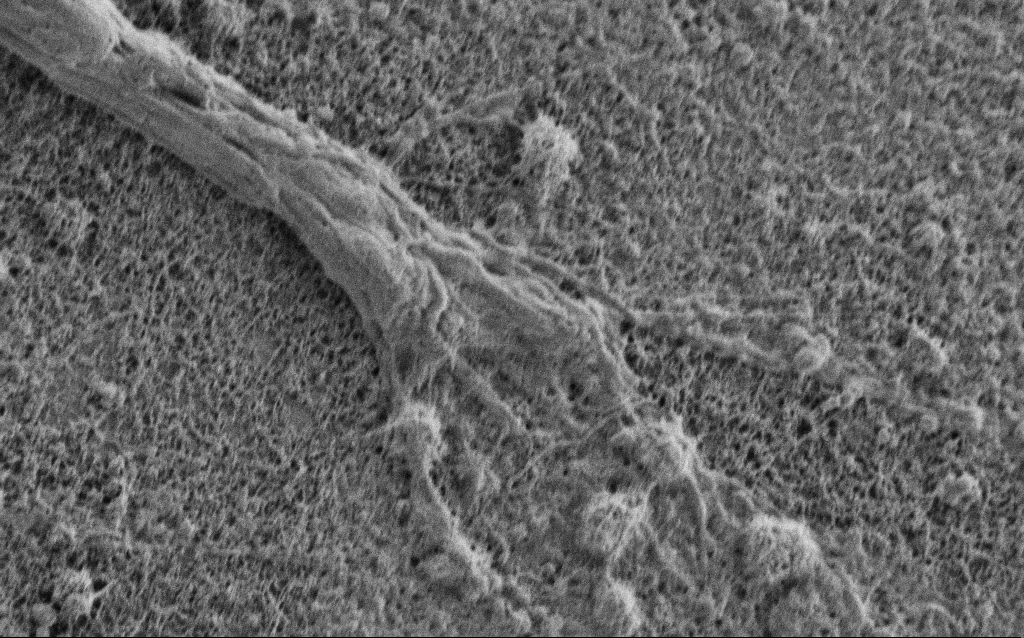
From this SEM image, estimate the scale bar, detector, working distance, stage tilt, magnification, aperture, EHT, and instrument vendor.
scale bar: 2000 nm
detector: SE2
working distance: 5.3 mm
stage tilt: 0°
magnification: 15 K X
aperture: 30 µm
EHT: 0.75 kV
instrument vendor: Zeiss